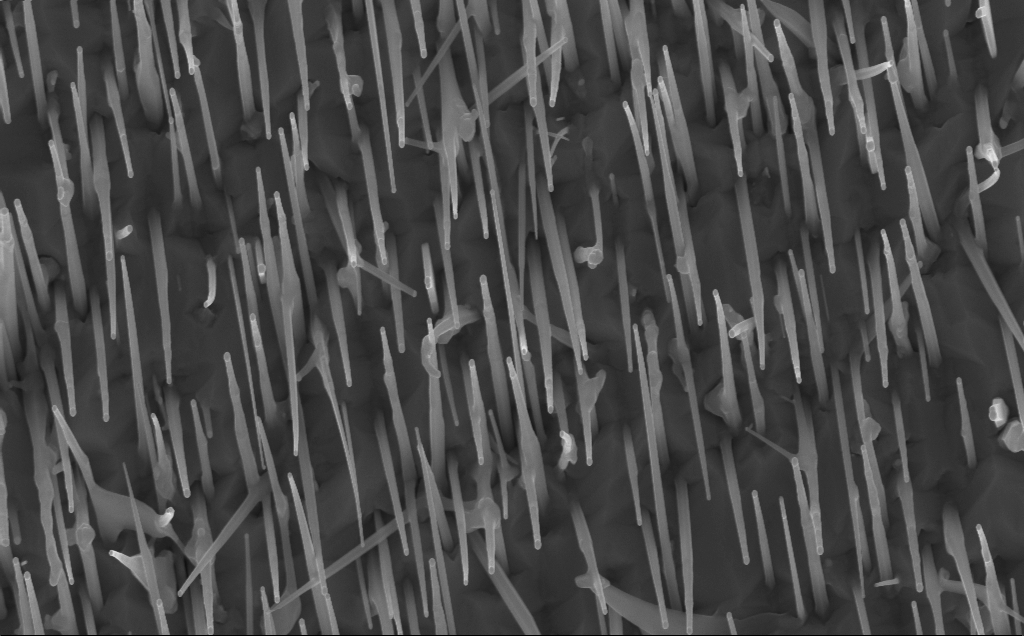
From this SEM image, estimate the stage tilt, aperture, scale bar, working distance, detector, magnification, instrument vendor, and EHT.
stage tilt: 0°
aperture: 30 µm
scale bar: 1000 nm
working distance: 7 mm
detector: InLens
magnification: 40 K X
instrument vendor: Zeiss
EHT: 10 kV